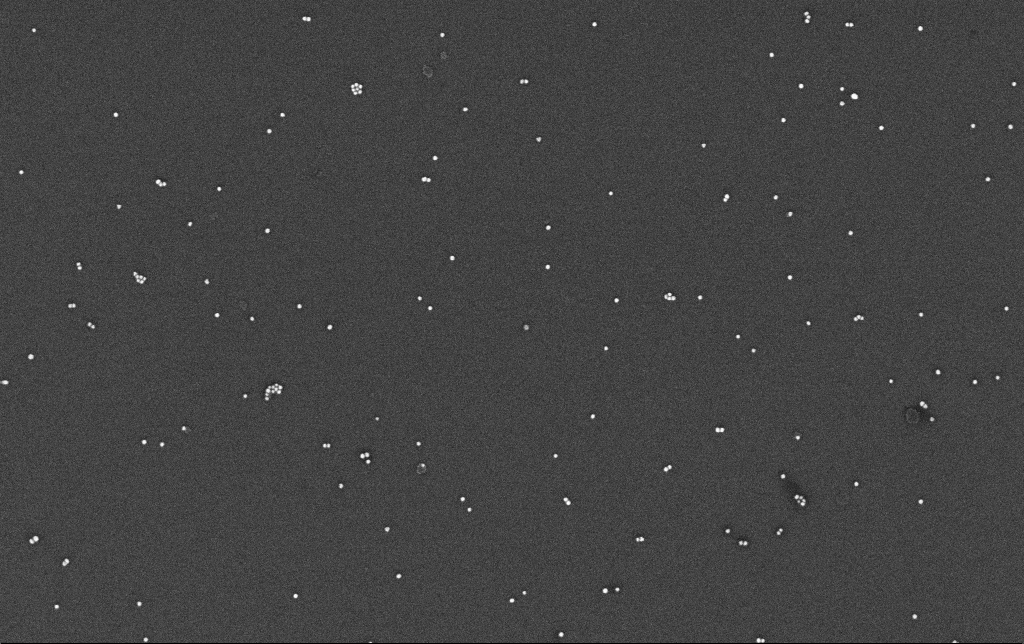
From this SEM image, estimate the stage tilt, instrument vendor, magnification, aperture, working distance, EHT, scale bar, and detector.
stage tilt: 0°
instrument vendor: Zeiss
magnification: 100 K X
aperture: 30 µm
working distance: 3.3 mm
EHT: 10 kV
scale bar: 200 nm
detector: InLens